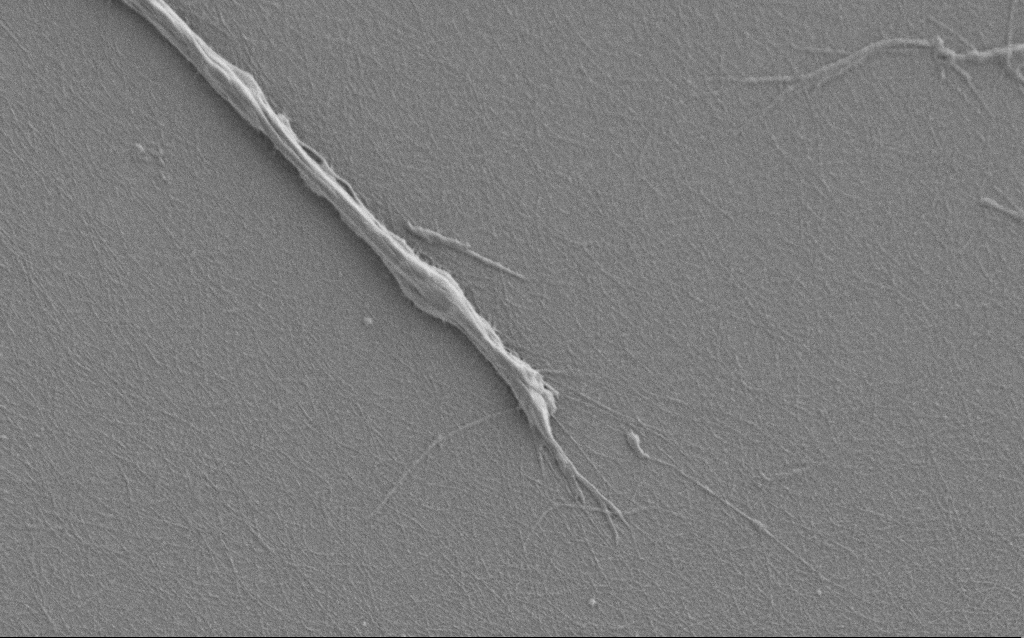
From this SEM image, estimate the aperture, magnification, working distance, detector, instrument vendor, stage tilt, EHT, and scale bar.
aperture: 30 µm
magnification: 7.5 K X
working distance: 6 mm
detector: SE2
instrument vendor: Zeiss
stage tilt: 0°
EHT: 1 kV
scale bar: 2000 nm